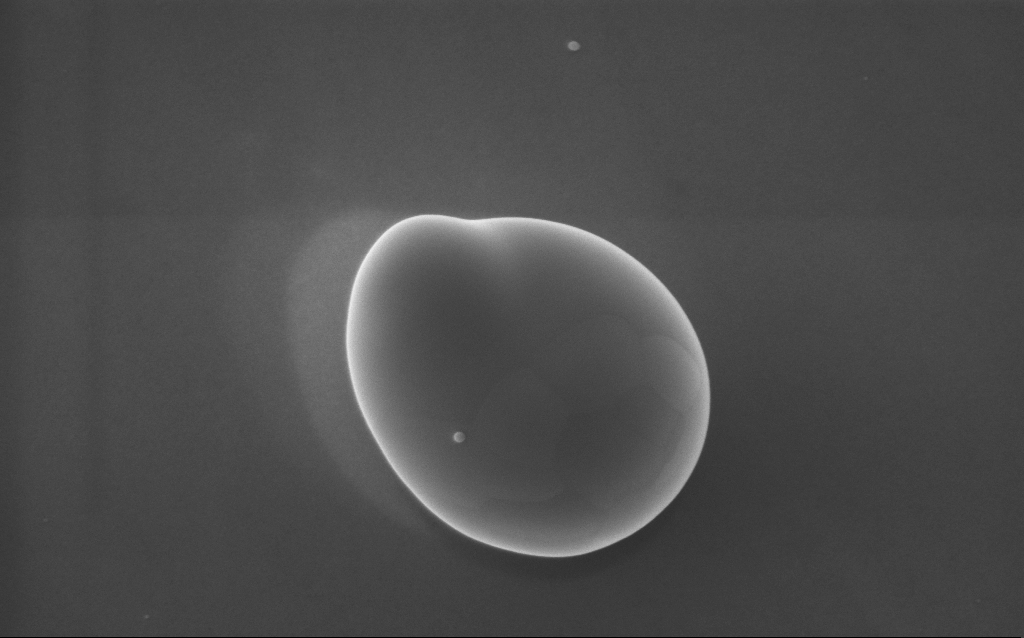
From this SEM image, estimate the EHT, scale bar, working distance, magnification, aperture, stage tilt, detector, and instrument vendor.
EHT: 5 kV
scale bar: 200 nm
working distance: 3 mm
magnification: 80 K X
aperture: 30 µm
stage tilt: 0°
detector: InLens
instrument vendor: Zeiss